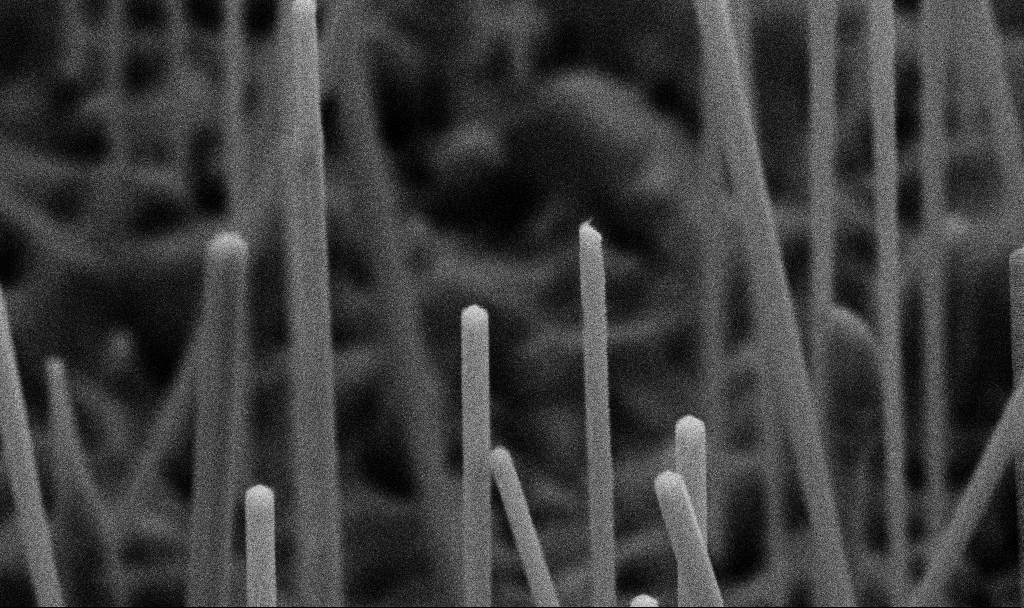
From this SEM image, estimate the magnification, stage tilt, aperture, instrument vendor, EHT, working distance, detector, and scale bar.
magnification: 82.25 K X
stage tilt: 45°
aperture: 30 µm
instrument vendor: Zeiss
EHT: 5 kV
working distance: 7.2 mm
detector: SE2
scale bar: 200 nm